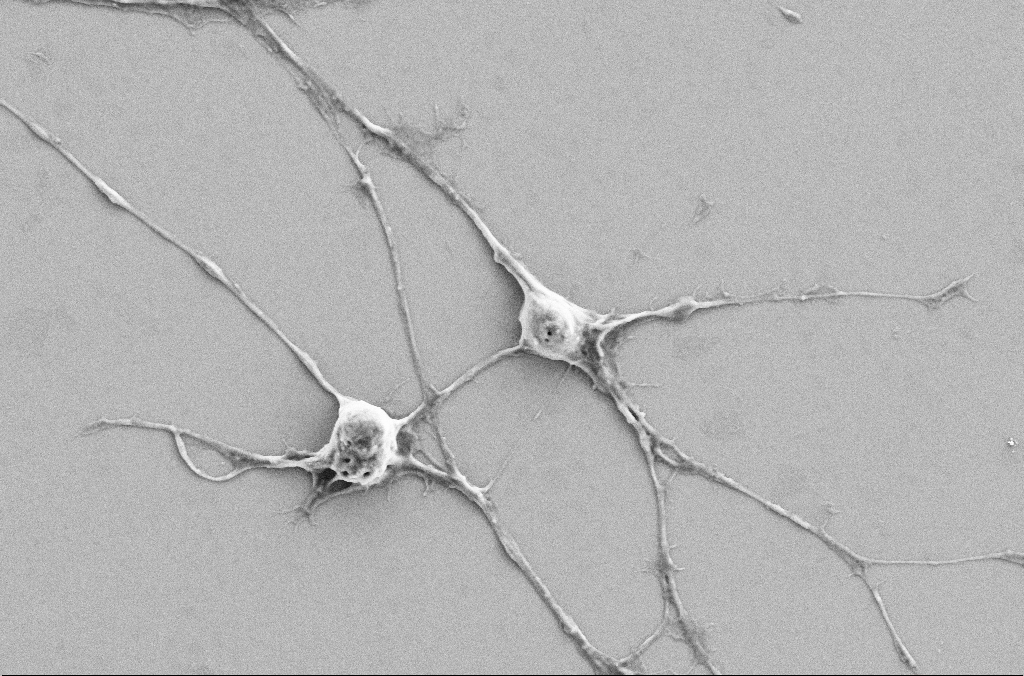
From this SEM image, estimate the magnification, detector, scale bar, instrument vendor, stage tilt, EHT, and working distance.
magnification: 5 K X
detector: SE2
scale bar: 10000 nm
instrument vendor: Zeiss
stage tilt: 0°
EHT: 5 kV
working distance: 3.2 mm